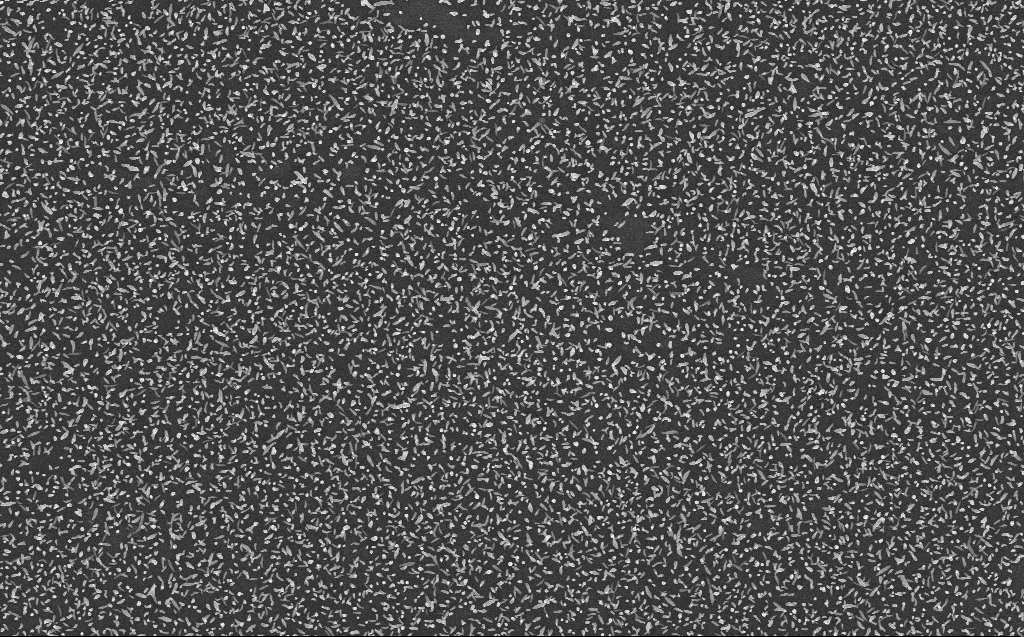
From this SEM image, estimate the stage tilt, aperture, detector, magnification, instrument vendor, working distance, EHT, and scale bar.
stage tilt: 0°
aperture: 30 µm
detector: InLens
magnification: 10 K X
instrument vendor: Zeiss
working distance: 3 mm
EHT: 10 kV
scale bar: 2000 nm